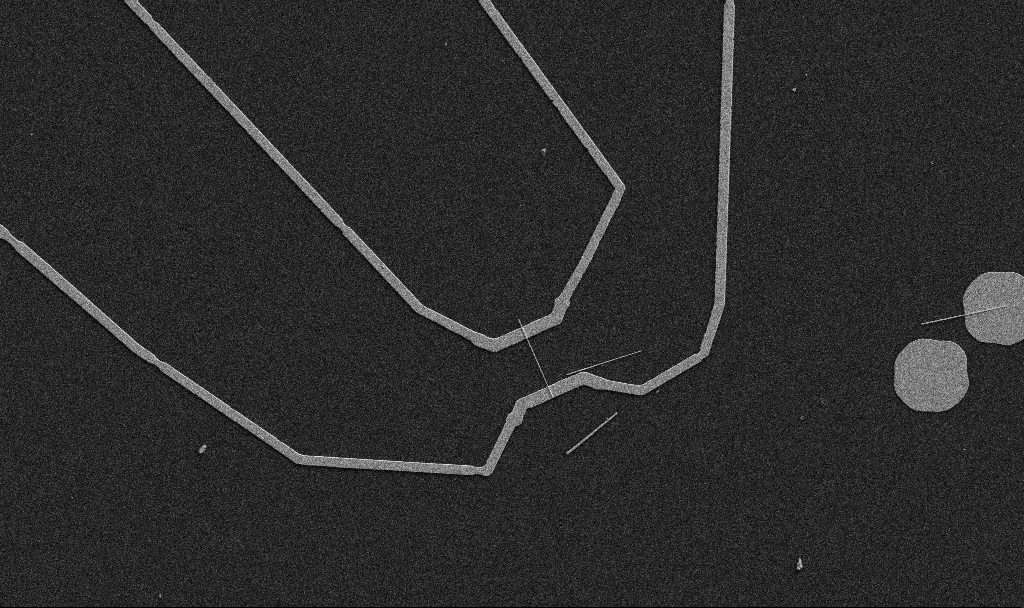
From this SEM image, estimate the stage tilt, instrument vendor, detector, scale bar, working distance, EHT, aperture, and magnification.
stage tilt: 0°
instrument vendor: Zeiss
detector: SE2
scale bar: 10000 nm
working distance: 10.7 mm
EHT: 5 kV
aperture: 30 µm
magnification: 5 K X